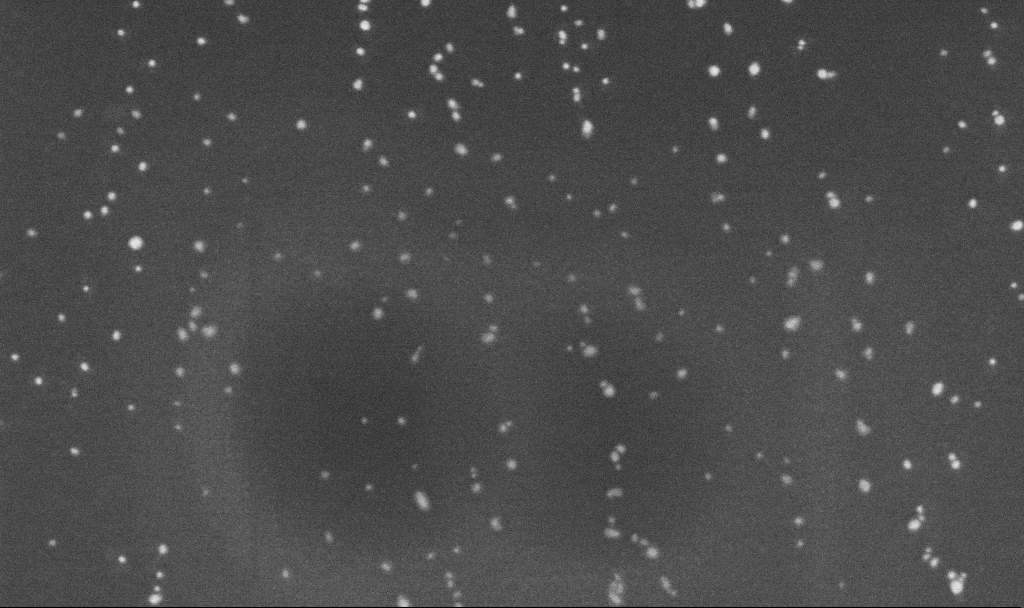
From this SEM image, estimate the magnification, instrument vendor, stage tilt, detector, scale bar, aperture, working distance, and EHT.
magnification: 200 K X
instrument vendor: Zeiss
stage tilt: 0°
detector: InLens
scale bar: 100 nm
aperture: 30 µm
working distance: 3.2 mm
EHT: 5 kV